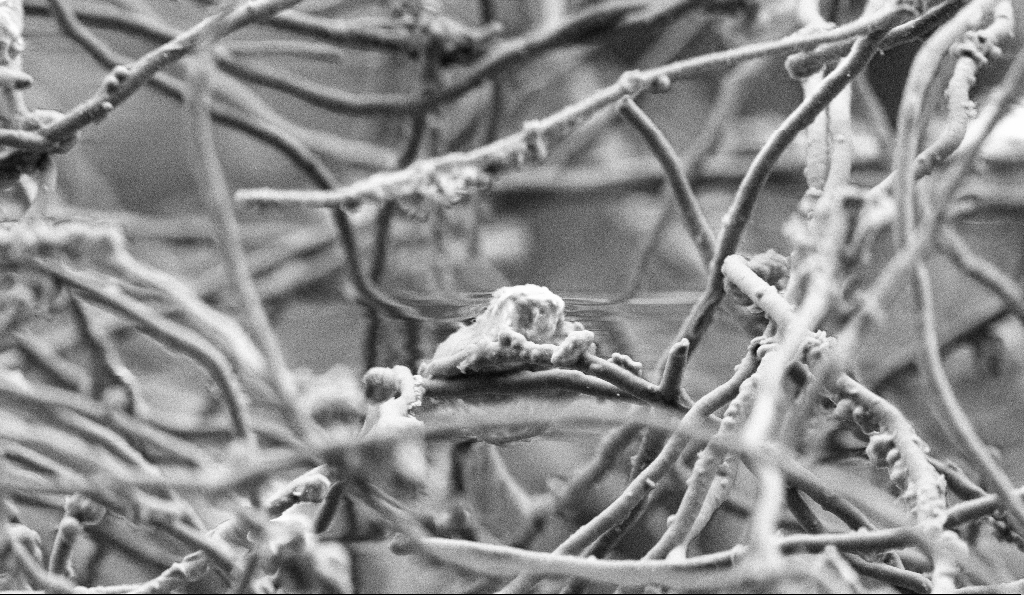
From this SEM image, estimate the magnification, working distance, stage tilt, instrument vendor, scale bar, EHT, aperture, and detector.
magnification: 10 K X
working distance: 5 mm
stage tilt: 0°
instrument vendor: Zeiss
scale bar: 2000 nm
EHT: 3 kV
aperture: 30 µm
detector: SE2